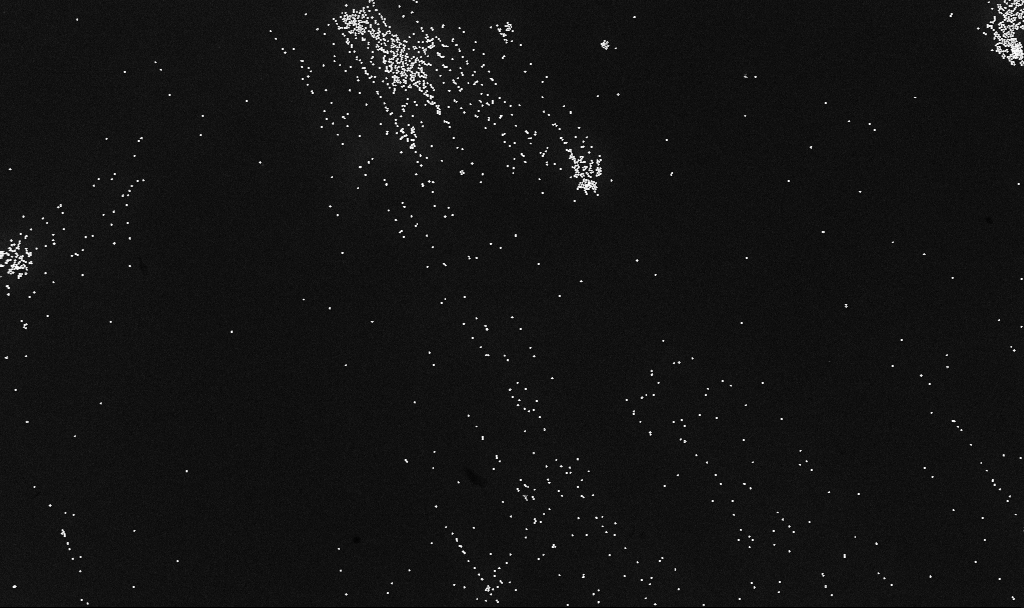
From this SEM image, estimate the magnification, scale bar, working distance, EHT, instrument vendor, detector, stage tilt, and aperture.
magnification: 12.72 K X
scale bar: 2000 nm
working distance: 3.4 mm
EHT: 10 kV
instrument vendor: Zeiss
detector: InLens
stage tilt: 0°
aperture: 30 µm